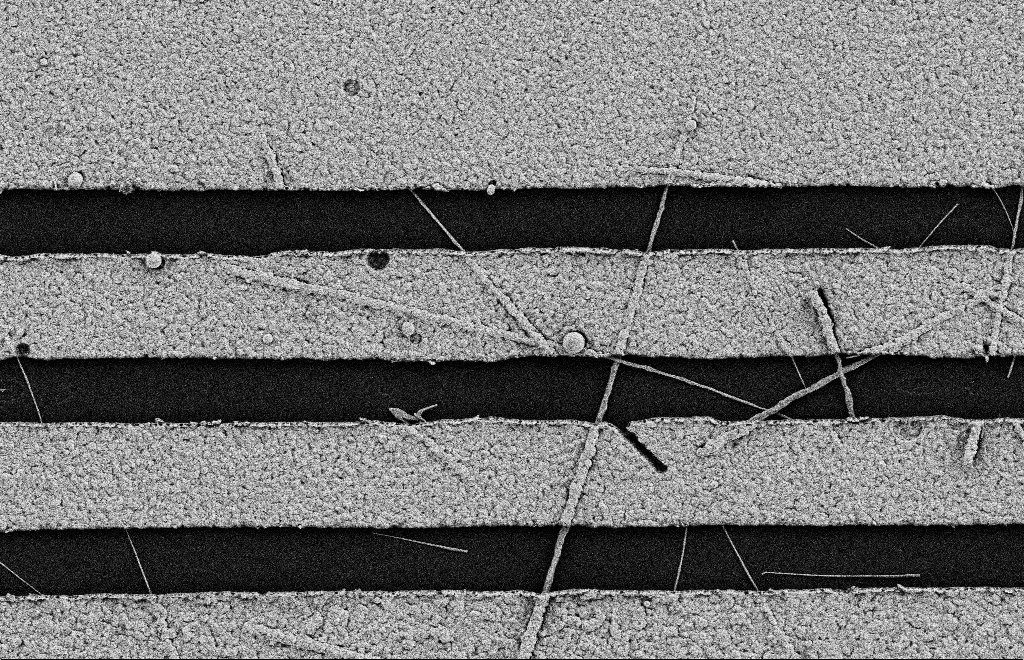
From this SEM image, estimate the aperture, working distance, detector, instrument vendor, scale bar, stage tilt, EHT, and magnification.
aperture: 20 µm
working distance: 11 mm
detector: SE2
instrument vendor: Zeiss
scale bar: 2000 nm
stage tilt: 0°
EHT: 2 kV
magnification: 15.45 K X